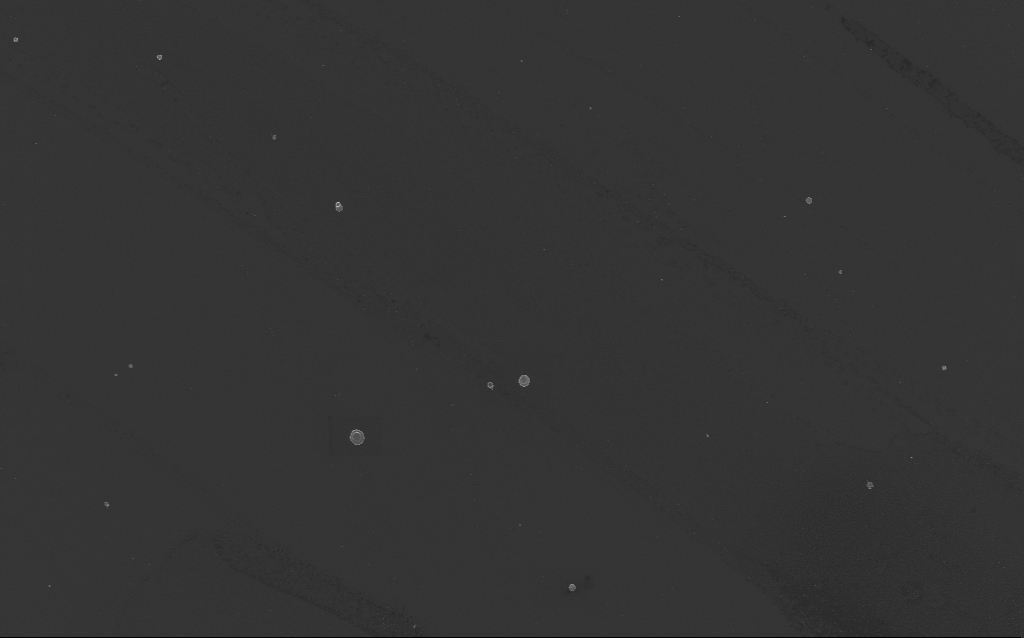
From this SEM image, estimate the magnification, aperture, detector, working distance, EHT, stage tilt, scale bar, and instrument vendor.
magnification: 2.46 K X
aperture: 30 µm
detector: InLens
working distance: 4 mm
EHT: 3 kV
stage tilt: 0°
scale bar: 10000 nm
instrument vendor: Zeiss